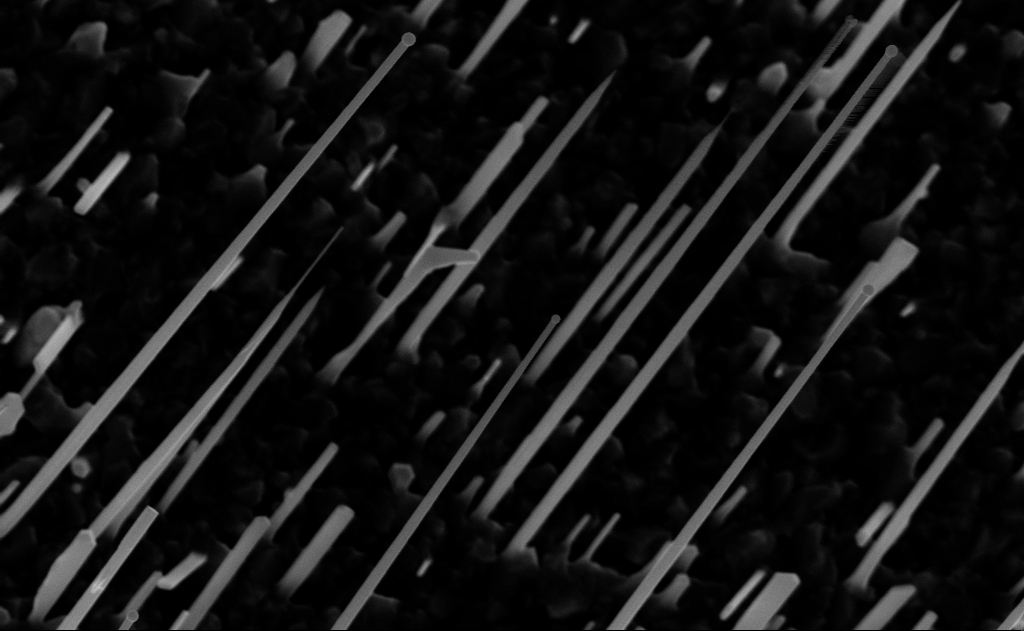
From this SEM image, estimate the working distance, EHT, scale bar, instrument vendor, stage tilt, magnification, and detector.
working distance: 10 mm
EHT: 10 kV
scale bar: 1000 nm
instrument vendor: Zeiss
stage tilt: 0°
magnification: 40 K X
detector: InLens